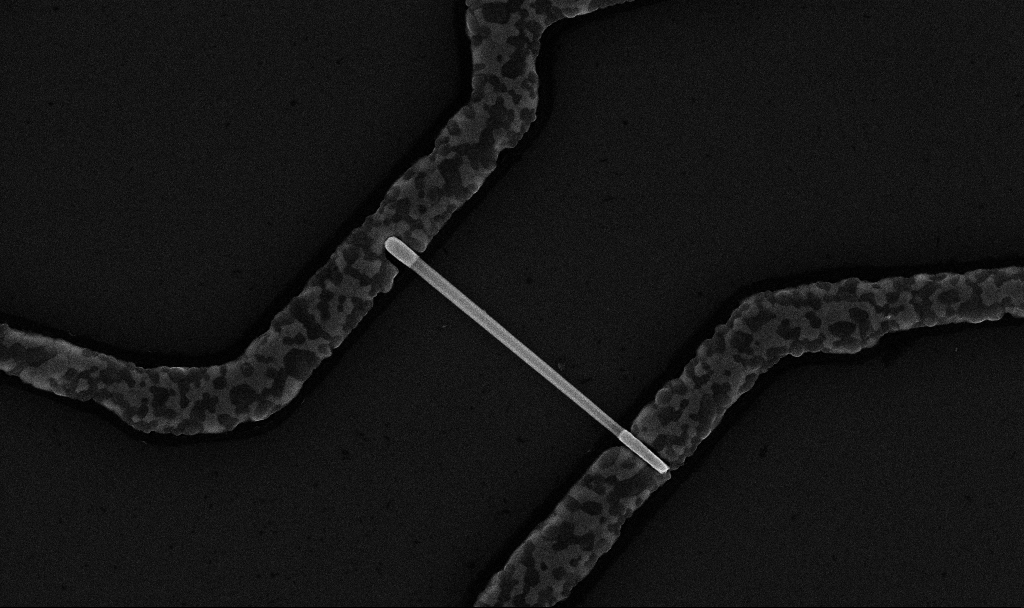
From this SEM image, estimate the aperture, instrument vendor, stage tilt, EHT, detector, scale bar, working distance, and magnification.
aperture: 30 µm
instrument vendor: Zeiss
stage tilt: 0°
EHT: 10 kV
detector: InLens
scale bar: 2000 nm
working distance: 6.7 mm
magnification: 28.68 K X